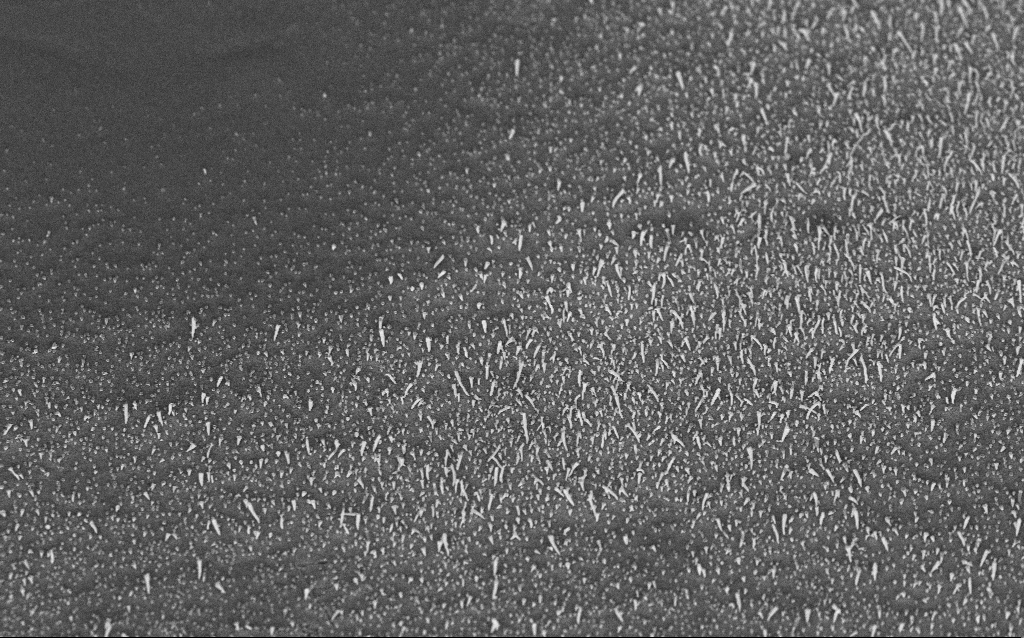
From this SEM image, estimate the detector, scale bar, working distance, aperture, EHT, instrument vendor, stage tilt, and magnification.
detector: InLens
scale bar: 2000 nm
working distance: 6.7 mm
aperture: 30 µm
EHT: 5 kV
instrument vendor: Zeiss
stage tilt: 45°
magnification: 10 K X